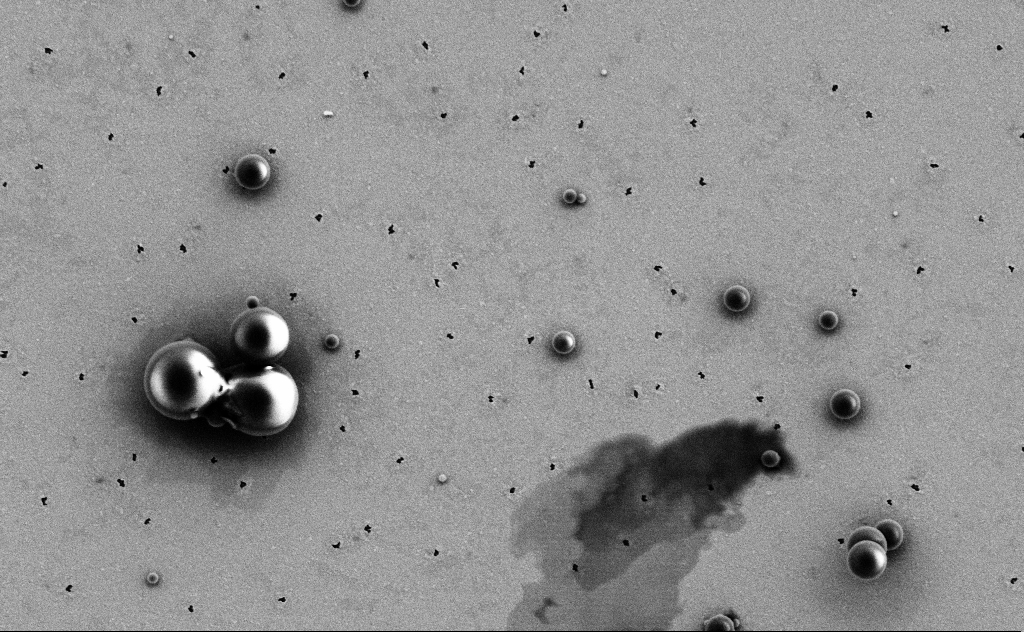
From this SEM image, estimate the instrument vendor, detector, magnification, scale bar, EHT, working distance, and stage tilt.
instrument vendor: Zeiss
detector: SE2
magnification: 12.18 K X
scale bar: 1000 nm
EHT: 3 kV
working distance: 11 mm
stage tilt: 0°